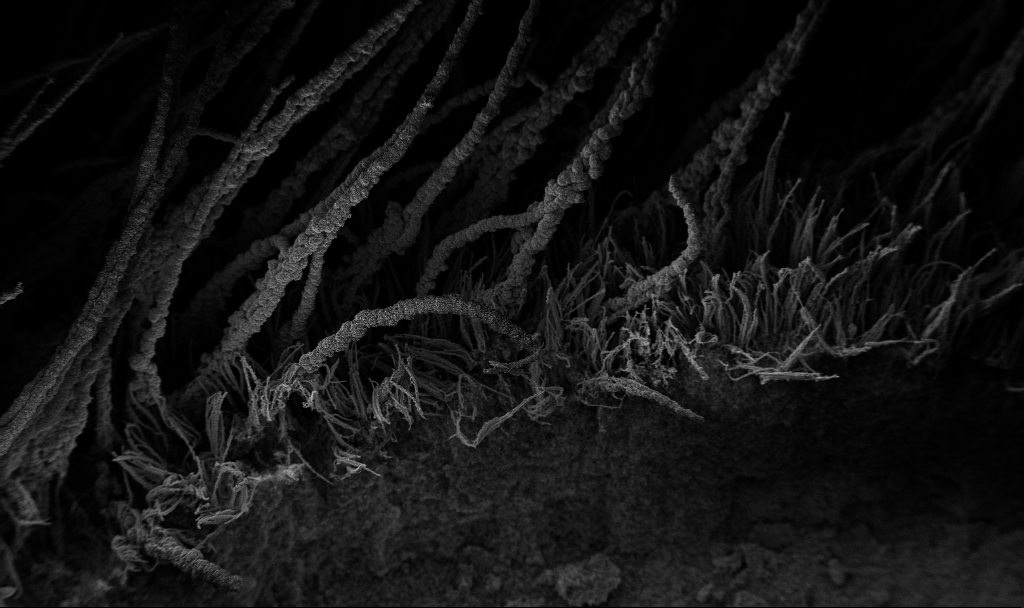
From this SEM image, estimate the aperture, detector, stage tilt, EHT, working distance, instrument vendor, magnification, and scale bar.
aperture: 30 µm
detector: InLens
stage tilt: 37.7°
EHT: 3 kV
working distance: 7.5 mm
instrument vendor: Zeiss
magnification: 0.15 K X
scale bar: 100000 nm